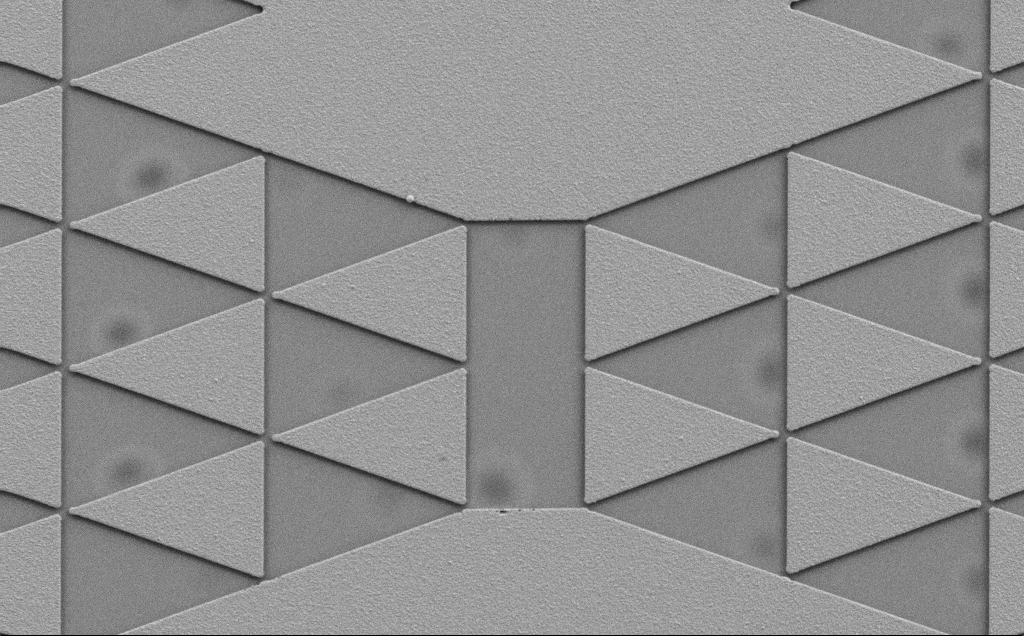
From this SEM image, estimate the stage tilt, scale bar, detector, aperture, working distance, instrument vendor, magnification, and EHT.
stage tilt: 0°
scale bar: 20000 nm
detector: SE2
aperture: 30 µm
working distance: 6 mm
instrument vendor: Zeiss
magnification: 0.883 K X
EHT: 5 kV